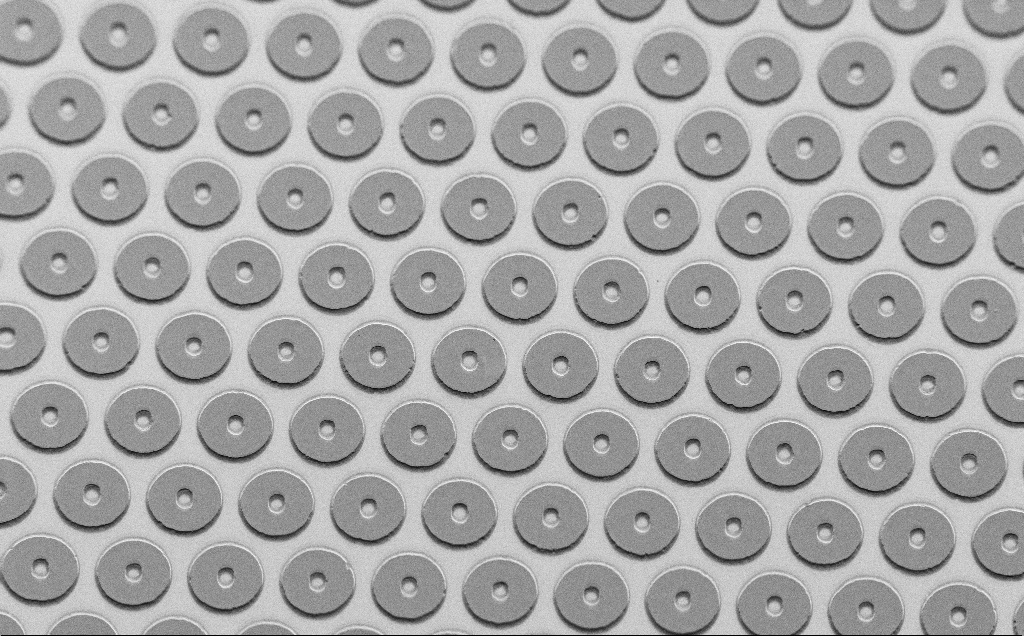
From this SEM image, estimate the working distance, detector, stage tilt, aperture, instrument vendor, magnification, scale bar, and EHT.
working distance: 7 mm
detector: SE2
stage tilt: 45°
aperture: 30 µm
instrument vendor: Zeiss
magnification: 0.561 K X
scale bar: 100000 nm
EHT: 1.5 kV